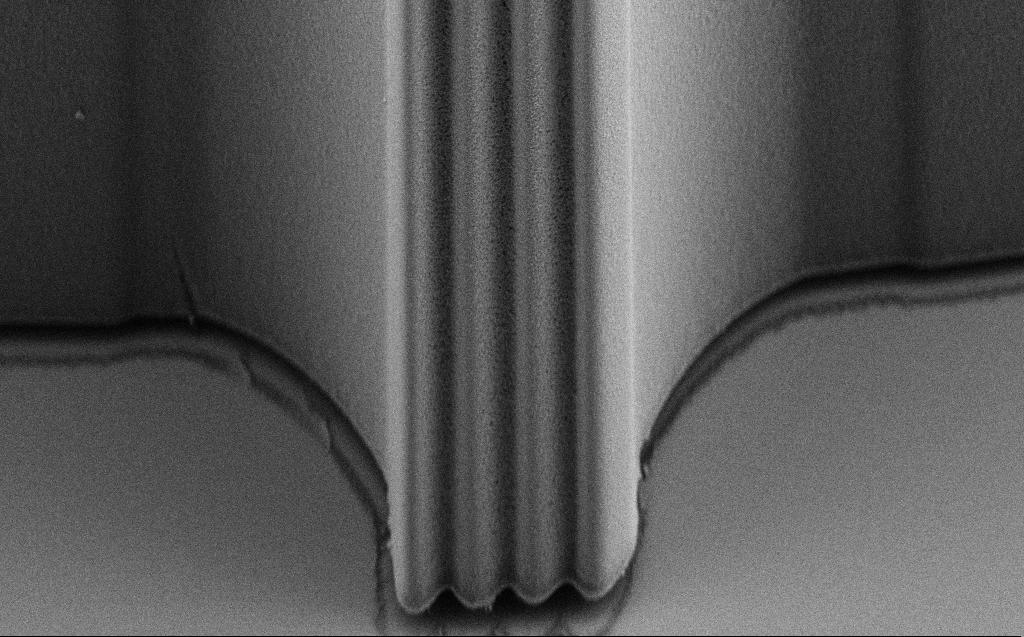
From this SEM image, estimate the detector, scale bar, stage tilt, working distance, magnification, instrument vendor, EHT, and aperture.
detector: SE2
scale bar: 20000 nm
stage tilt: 45°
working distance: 7 mm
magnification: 1.97 K X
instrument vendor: Zeiss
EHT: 5 kV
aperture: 30 µm